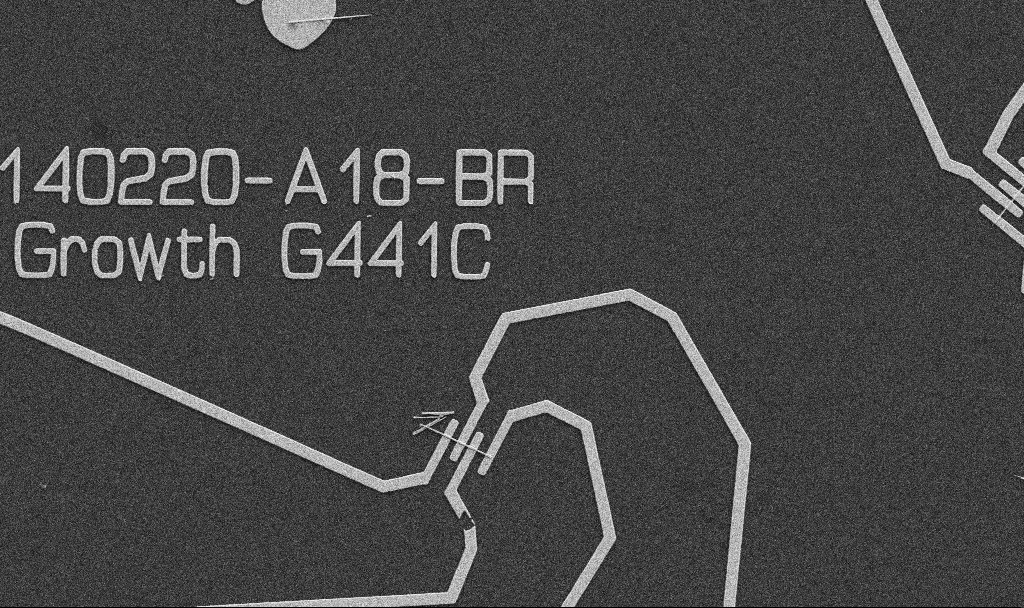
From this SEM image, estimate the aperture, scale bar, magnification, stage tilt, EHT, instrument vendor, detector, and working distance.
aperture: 30 µm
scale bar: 10000 nm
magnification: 5 K X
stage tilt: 0°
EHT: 5 kV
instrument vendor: Zeiss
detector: SE2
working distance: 10.7 mm